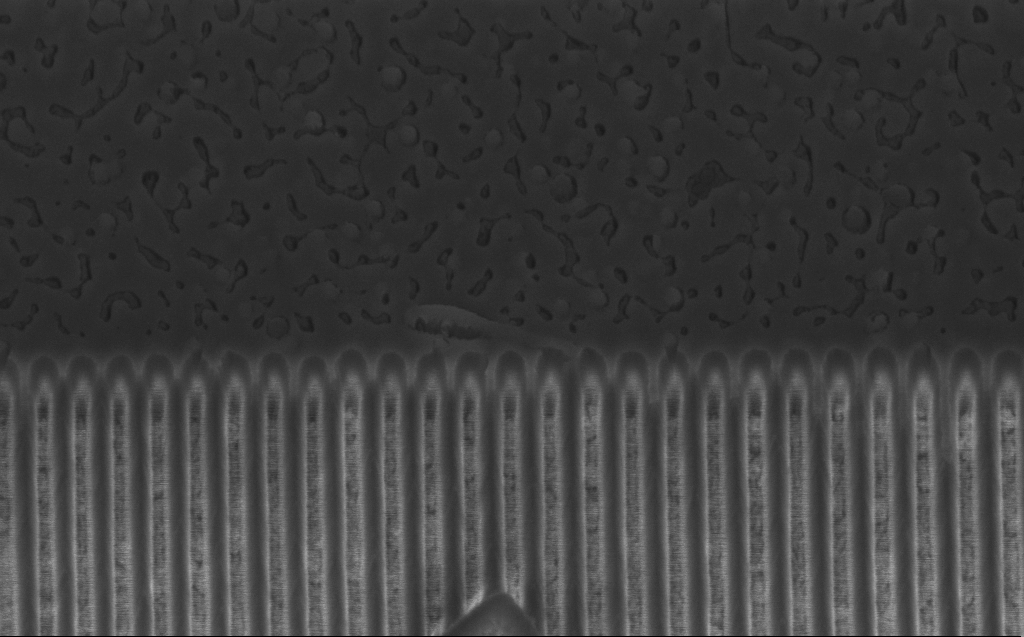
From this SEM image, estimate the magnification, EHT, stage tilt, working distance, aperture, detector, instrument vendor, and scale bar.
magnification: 50.89 K X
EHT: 3 kV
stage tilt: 45°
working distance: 6 mm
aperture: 30 µm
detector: InLens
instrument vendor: Zeiss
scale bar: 1000 nm